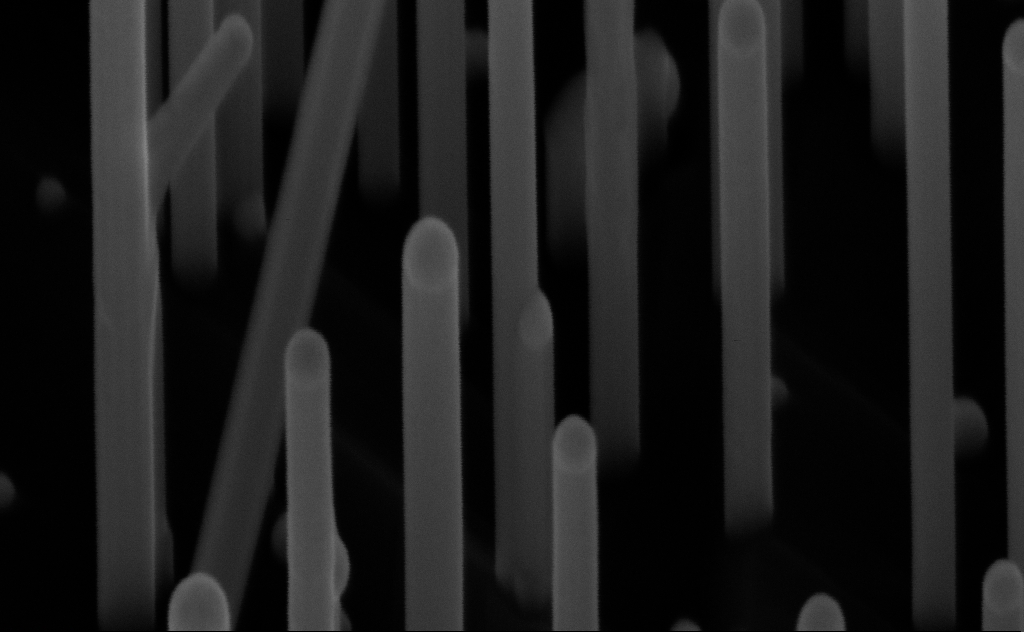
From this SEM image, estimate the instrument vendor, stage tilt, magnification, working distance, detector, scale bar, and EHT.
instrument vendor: Zeiss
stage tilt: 45°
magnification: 311.25 K X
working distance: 7 mm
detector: InLens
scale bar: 200 nm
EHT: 10 kV